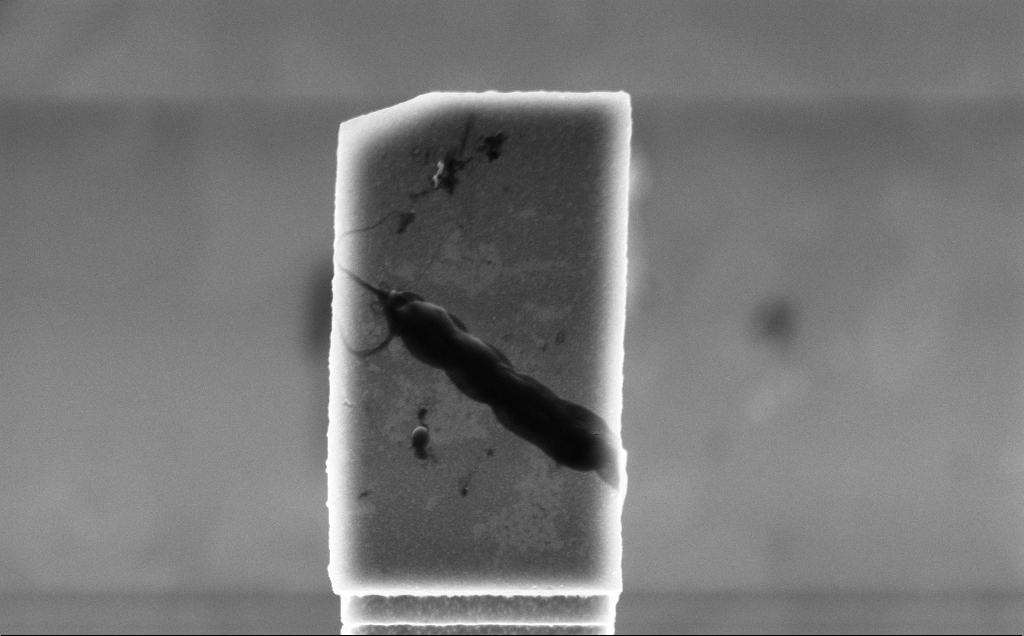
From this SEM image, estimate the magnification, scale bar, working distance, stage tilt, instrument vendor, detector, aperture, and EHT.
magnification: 37.11 K X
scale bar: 1000 nm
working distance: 7 mm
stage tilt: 45°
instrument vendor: Zeiss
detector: InLens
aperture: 30 µm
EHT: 5 kV